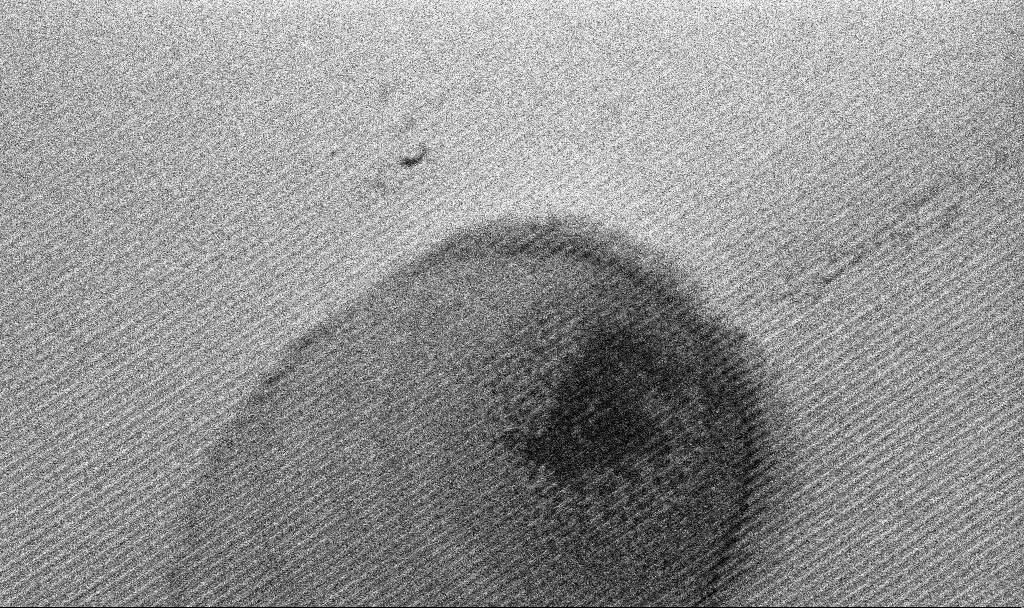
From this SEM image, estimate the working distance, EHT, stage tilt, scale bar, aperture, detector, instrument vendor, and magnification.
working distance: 8.5 mm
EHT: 10 kV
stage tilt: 45°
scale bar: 2000 nm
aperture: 60 µm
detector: InLens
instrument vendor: Zeiss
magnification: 9.42 K X